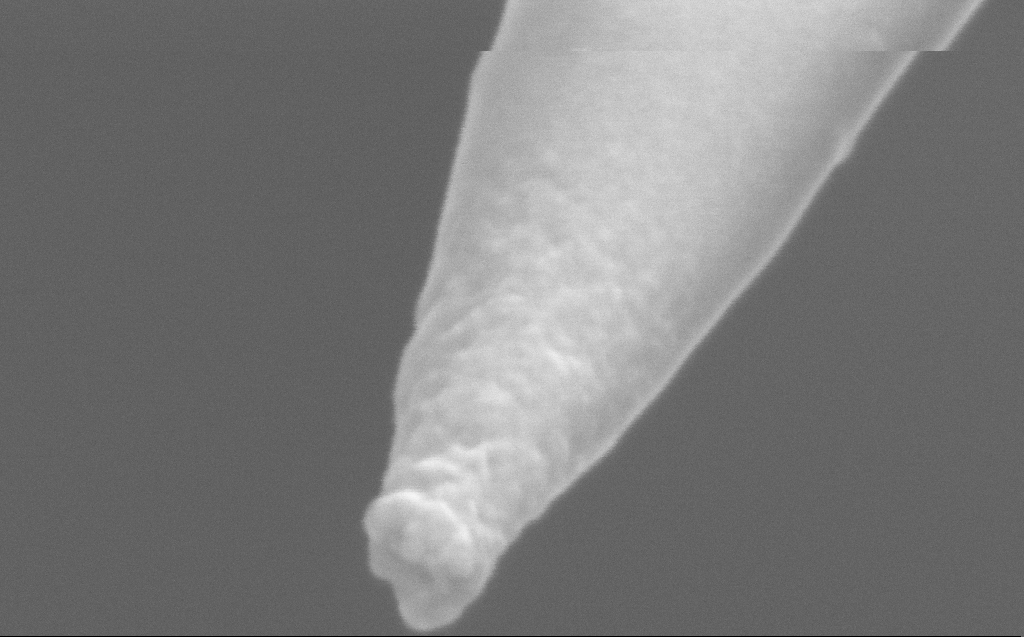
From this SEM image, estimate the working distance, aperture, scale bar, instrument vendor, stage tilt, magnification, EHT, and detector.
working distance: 6 mm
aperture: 30 µm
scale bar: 100 nm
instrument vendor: Zeiss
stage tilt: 45°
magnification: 500 K X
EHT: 5 kV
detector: InLens